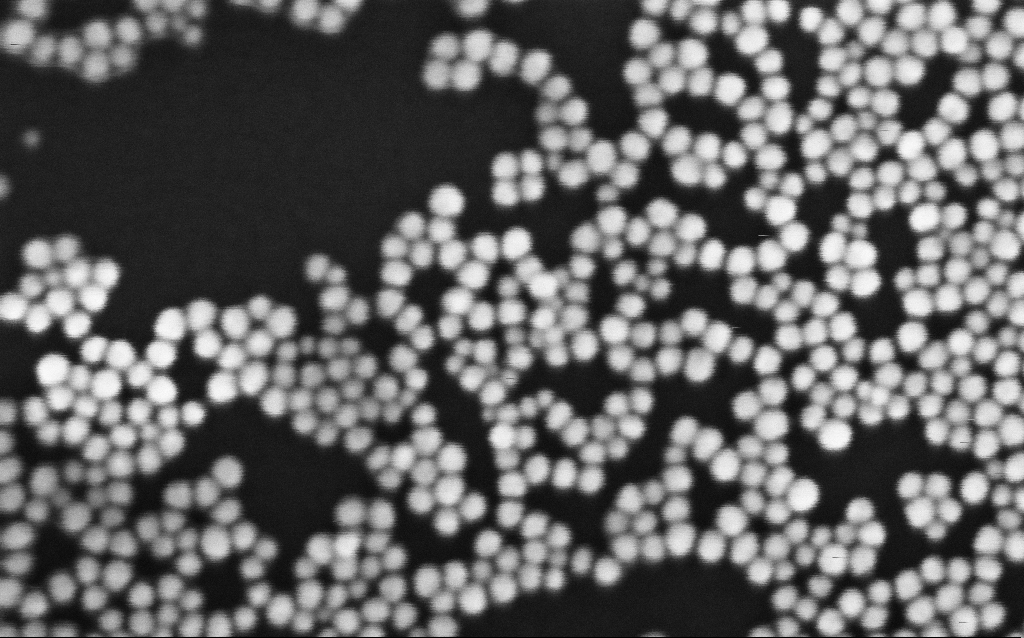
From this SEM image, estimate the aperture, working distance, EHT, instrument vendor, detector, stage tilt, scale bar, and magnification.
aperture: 30 µm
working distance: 3.3 mm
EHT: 5 kV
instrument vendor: Zeiss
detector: InLens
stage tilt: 0°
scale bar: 100 nm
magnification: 500 K X